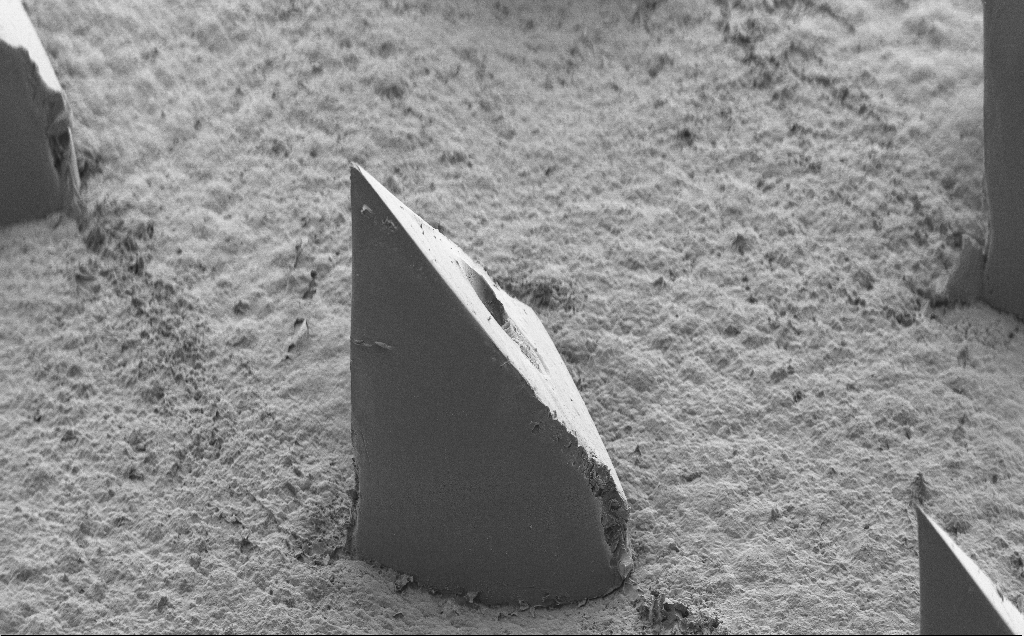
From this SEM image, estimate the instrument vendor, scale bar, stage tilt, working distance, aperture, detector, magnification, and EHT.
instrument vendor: Zeiss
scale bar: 100000 nm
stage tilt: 40°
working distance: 9 mm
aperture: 30 µm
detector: SE2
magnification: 0.174 K X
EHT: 5 kV